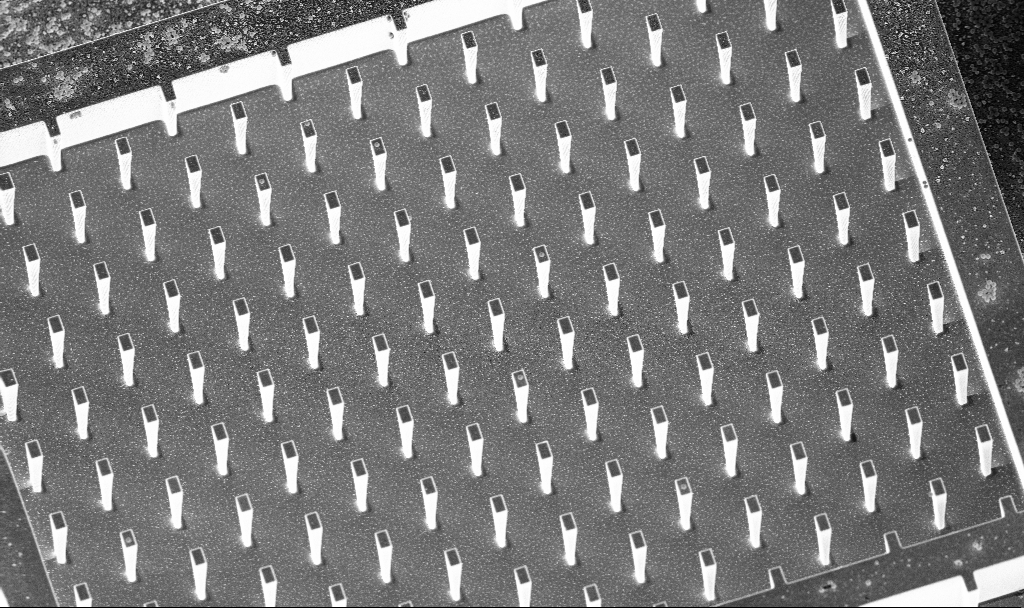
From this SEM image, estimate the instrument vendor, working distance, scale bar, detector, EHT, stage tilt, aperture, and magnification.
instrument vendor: Zeiss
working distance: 5.2 mm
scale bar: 10000 nm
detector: InLens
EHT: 5 kV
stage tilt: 20°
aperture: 30 µm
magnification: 2.4 K X